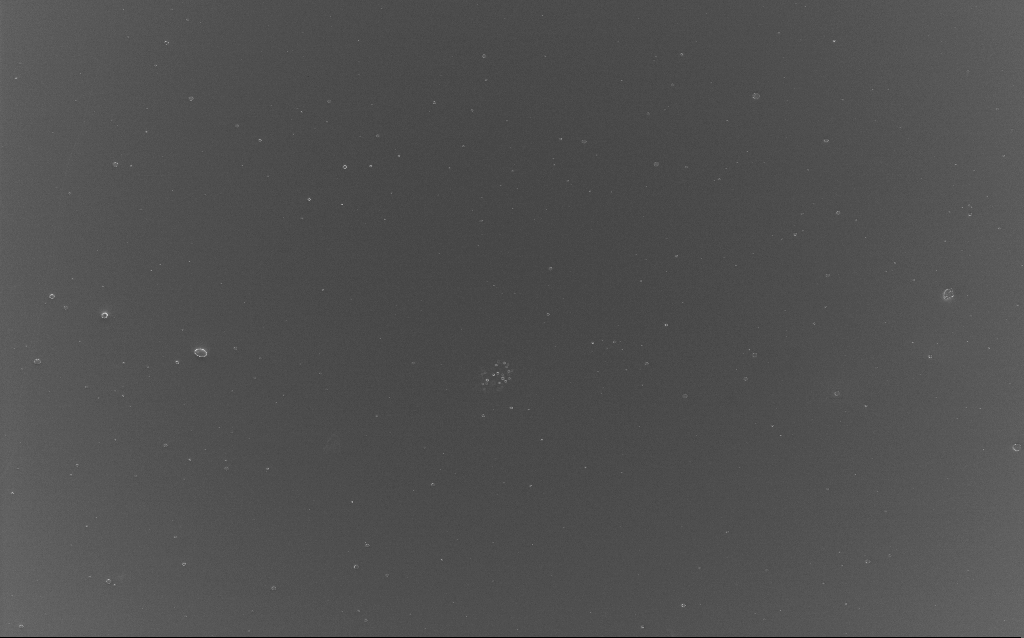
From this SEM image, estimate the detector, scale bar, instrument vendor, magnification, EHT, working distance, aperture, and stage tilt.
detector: InLens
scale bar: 20000 nm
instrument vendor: Zeiss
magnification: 0.989 K X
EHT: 5 kV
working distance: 2 mm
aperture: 30 µm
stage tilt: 0°